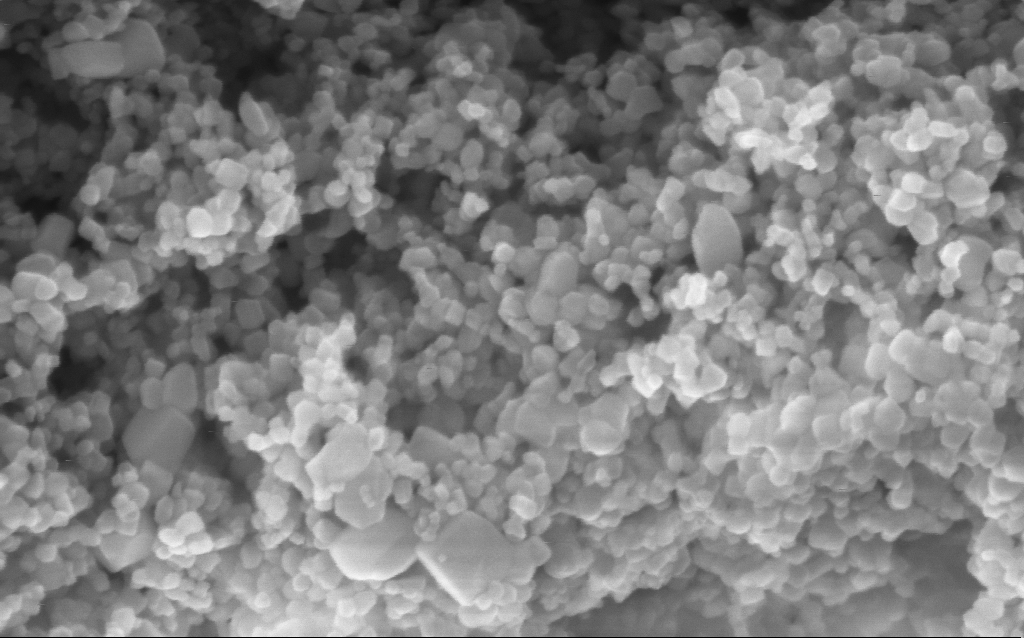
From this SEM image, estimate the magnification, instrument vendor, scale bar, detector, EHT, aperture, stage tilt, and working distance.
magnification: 294.05 K X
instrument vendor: Zeiss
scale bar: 200 nm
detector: InLens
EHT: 5 kV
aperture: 30 µm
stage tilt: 0°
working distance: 4.4 mm